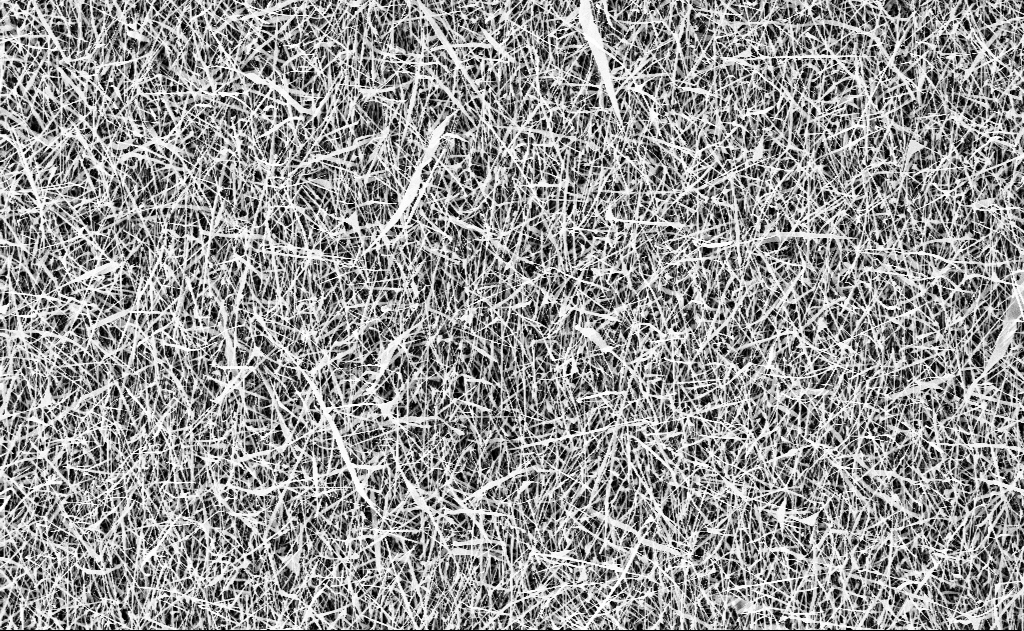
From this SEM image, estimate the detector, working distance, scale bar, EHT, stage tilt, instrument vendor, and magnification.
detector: InLens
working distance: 16 mm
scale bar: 10000 nm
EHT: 10 kV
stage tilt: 0°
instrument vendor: Zeiss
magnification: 5 K X